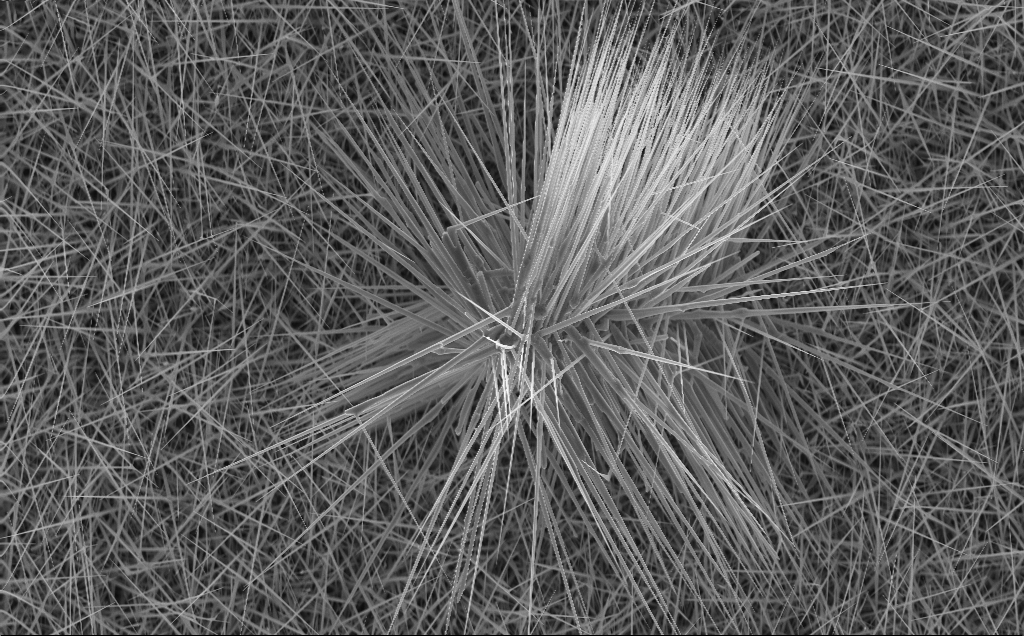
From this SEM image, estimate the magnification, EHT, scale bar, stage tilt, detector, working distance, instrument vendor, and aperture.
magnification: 3.6 K X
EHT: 10 kV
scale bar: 20000 nm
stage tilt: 0°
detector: InLens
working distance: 4 mm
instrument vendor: Zeiss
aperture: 30 µm